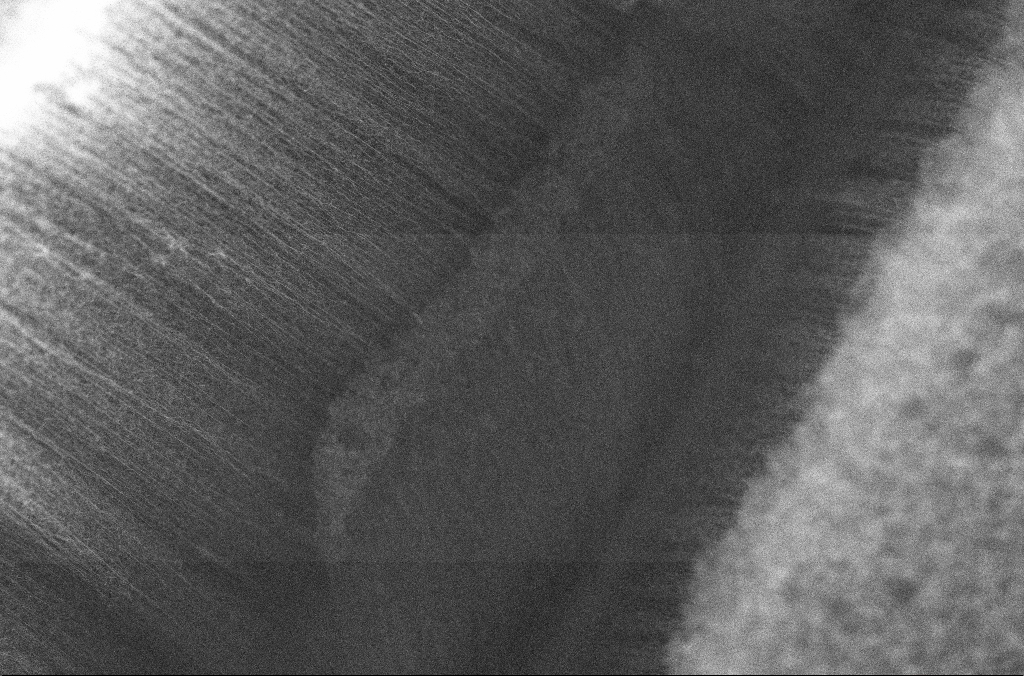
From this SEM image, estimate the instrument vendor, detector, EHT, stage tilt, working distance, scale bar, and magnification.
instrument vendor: Zeiss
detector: InLens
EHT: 10 kV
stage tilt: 0°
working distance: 4 mm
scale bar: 2000 nm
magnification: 10 K X